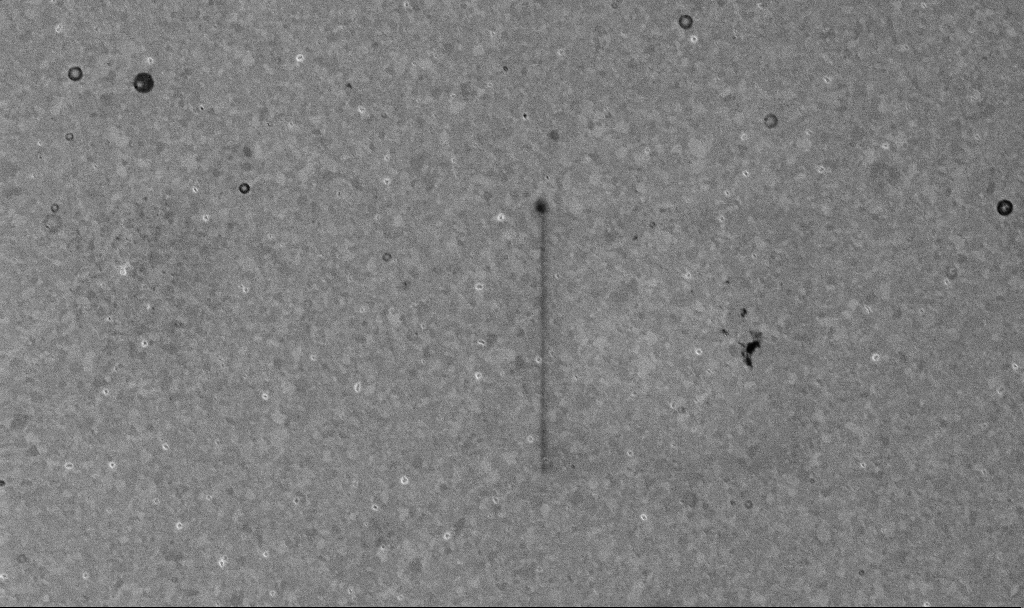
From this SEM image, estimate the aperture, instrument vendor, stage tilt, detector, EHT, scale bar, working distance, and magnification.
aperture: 30 µm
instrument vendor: Zeiss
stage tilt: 0°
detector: InLens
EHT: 10 kV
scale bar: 2000 nm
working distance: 3.3 mm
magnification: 10.25 K X